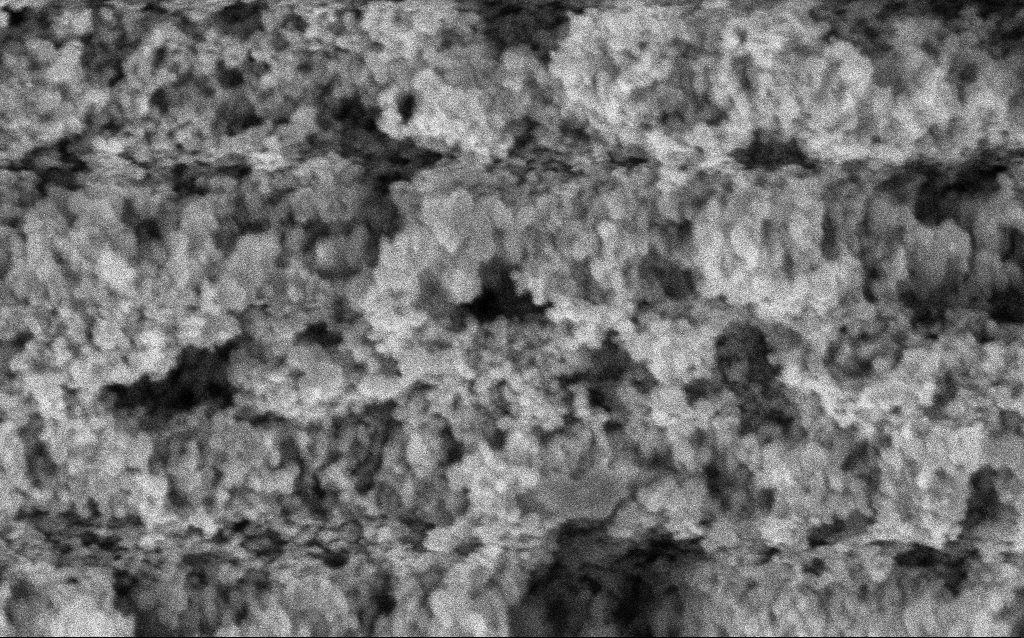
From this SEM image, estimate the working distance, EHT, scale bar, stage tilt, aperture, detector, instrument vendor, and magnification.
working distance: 10 mm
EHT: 3 kV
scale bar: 100 nm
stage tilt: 0°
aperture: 30 µm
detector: InLens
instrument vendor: Zeiss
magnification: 162.39 K X